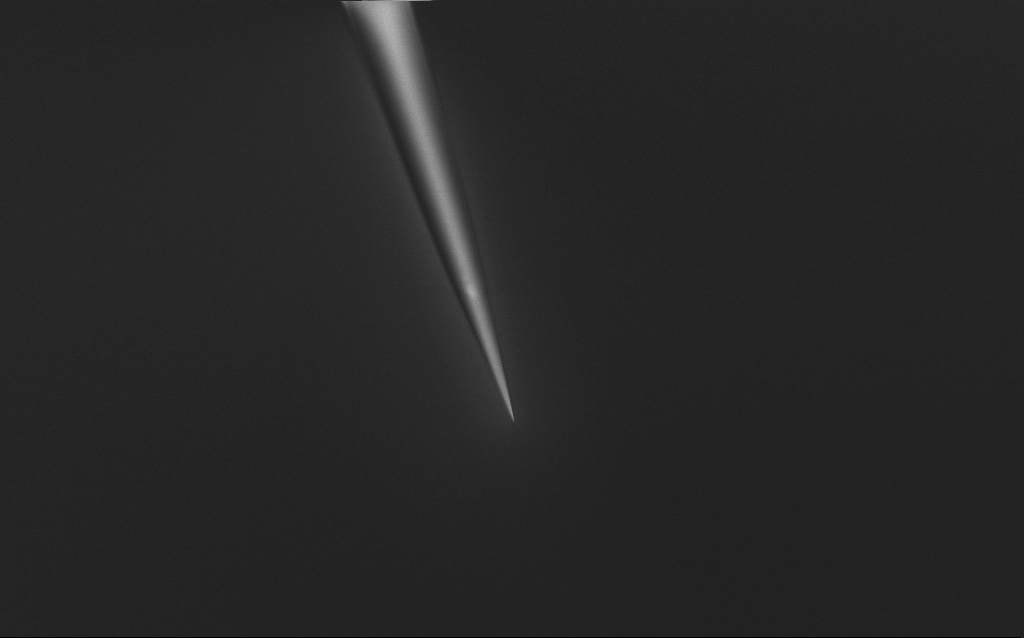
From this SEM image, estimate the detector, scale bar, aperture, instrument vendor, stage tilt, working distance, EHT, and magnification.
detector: InLens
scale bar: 20000 nm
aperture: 30 µm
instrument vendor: Zeiss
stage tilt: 45°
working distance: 7 mm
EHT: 1 kV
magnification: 1 K X